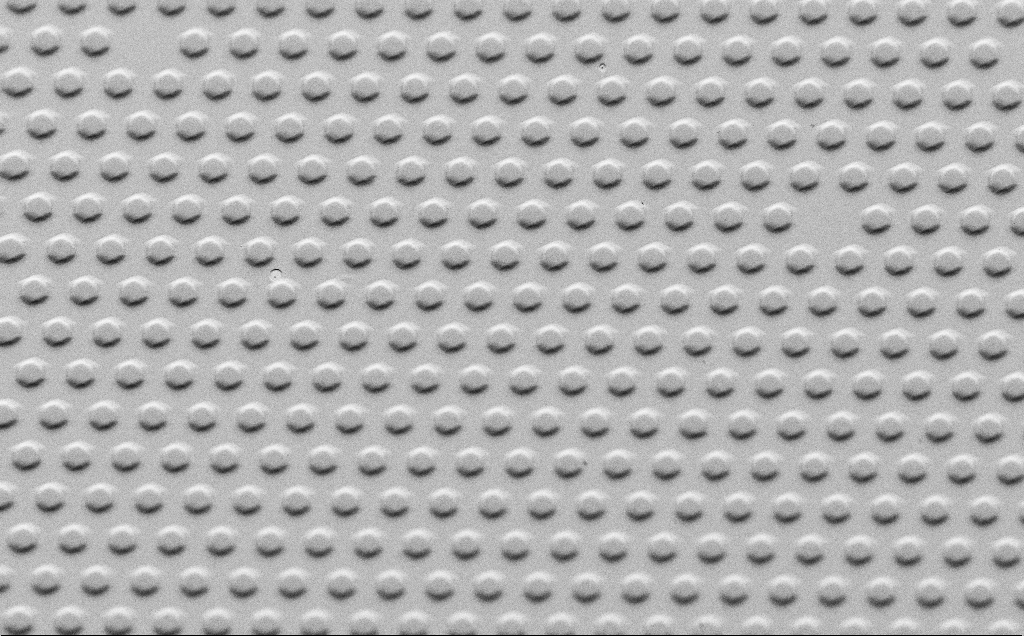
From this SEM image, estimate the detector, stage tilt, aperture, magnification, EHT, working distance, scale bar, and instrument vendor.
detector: SE2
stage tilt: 45°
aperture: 30 µm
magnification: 0.918 K X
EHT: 1.5 kV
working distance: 4 mm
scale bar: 20000 nm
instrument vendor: Zeiss